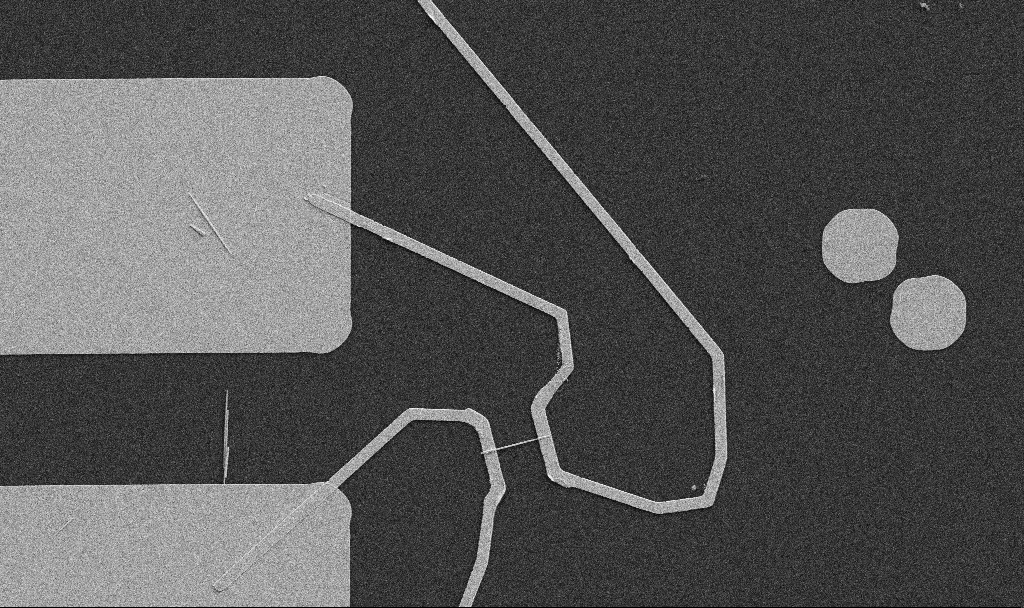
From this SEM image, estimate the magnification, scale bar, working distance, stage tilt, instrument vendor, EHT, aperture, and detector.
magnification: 5 K X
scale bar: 10000 nm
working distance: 10.7 mm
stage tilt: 0°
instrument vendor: Zeiss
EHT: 5 kV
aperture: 30 µm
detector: SE2